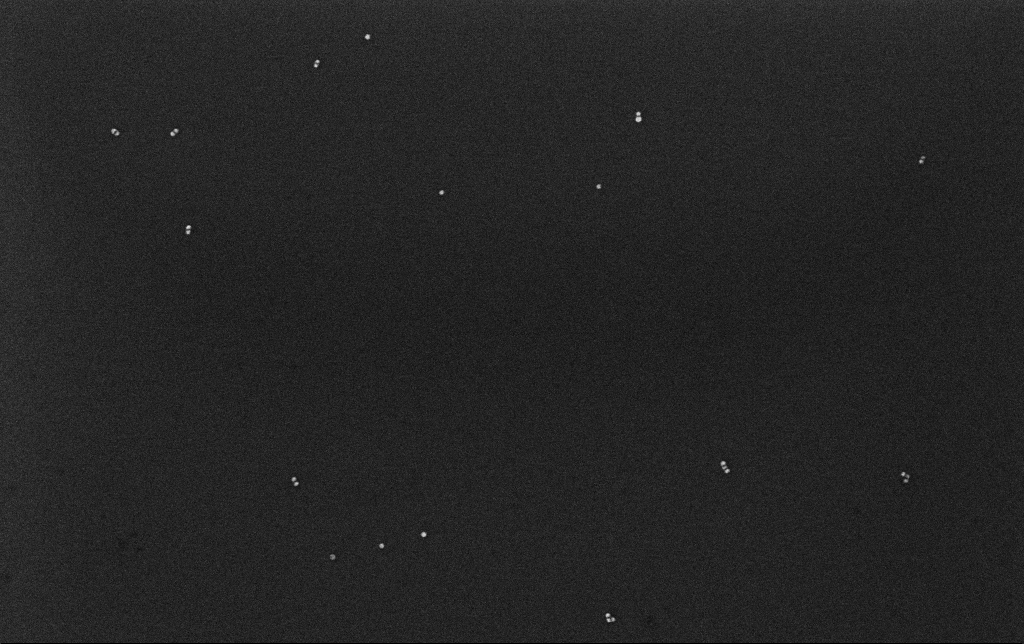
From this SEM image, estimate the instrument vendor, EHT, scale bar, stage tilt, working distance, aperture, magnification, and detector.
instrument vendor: Zeiss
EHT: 10 kV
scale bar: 200 nm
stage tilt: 0°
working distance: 3.2 mm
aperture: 30 µm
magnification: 100 K X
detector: InLens